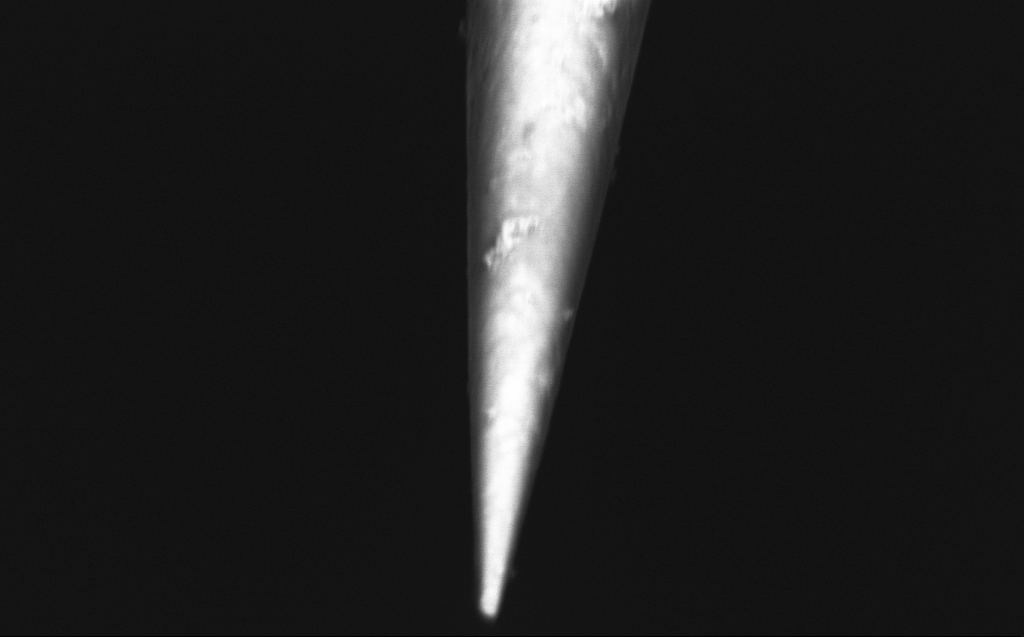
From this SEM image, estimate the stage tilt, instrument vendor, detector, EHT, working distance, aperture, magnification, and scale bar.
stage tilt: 45°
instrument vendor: Zeiss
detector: InLens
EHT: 1 kV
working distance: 5 mm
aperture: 30 µm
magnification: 50 K X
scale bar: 1000 nm